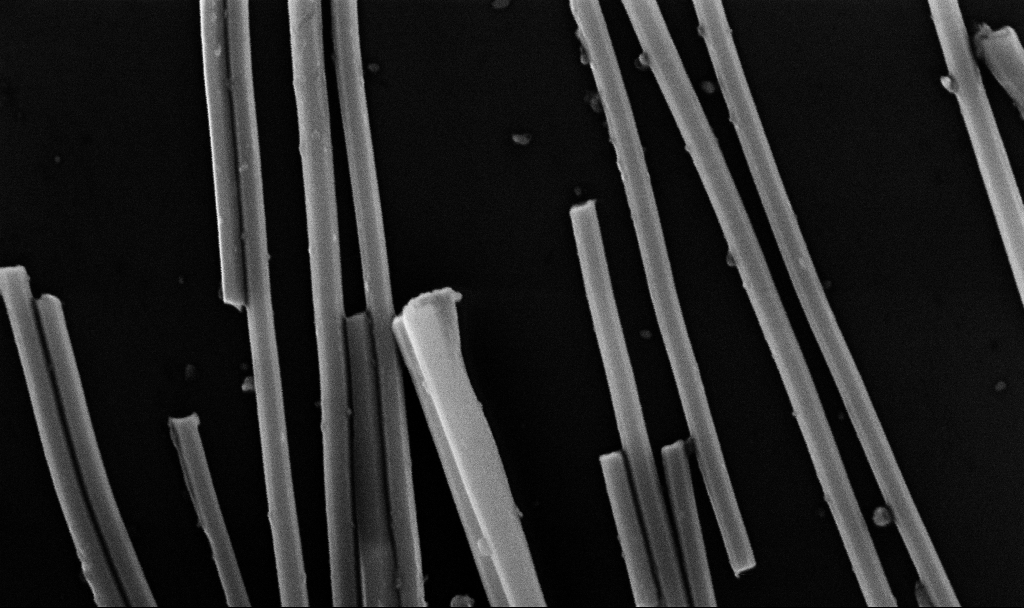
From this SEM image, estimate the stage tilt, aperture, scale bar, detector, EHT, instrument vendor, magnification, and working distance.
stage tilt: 0°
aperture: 30 µm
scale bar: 200 nm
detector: InLens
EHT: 10 kV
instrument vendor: Zeiss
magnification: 97.21 K X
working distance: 8.7 mm